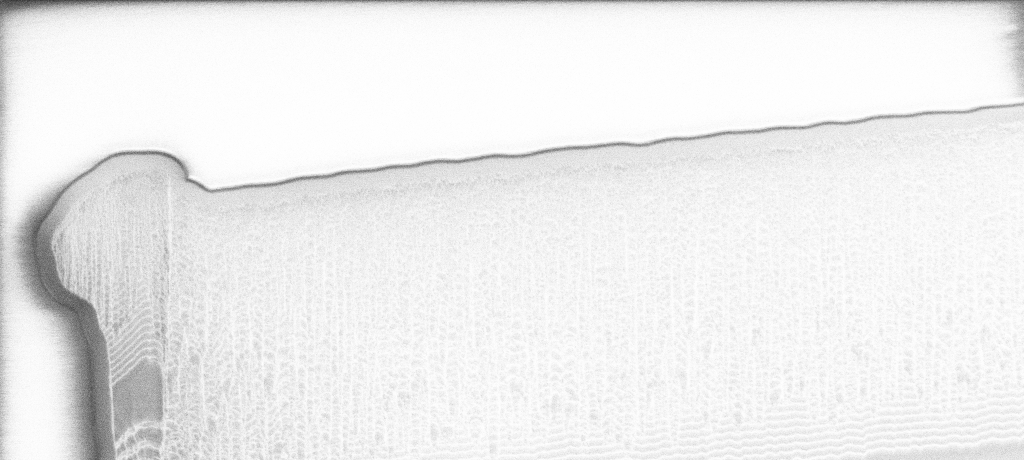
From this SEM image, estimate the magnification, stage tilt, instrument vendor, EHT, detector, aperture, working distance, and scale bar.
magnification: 8.06 K X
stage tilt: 45°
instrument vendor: Zeiss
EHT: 1.2 kV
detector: InLens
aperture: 30 µm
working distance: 7 mm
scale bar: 2000 nm